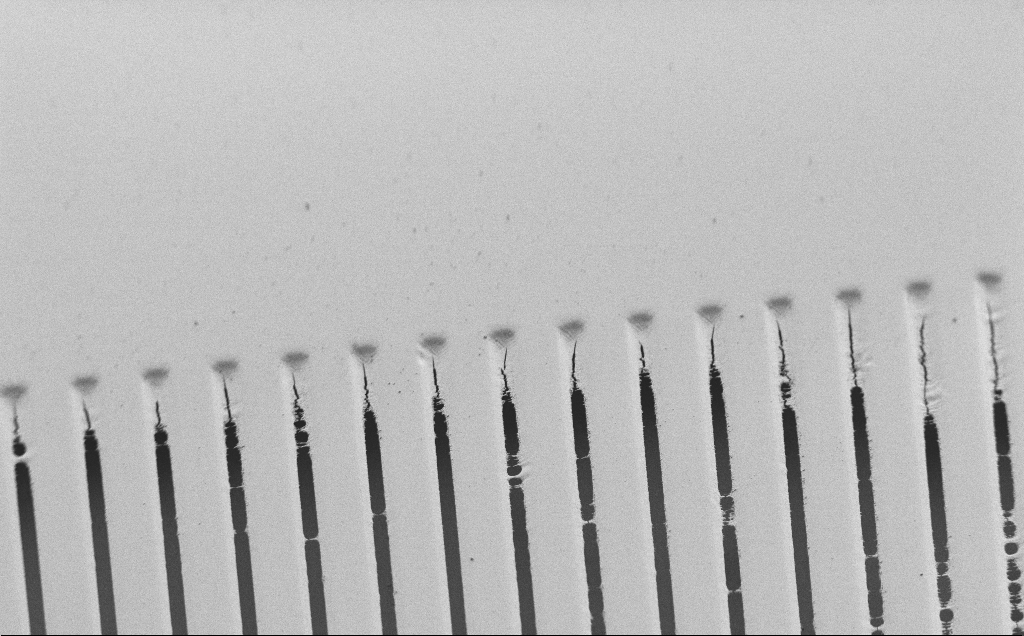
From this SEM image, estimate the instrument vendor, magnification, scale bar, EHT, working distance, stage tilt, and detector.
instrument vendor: Zeiss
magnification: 0.789 K X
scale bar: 20000 nm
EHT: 1.2 kV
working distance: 8 mm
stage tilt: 45°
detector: SE2